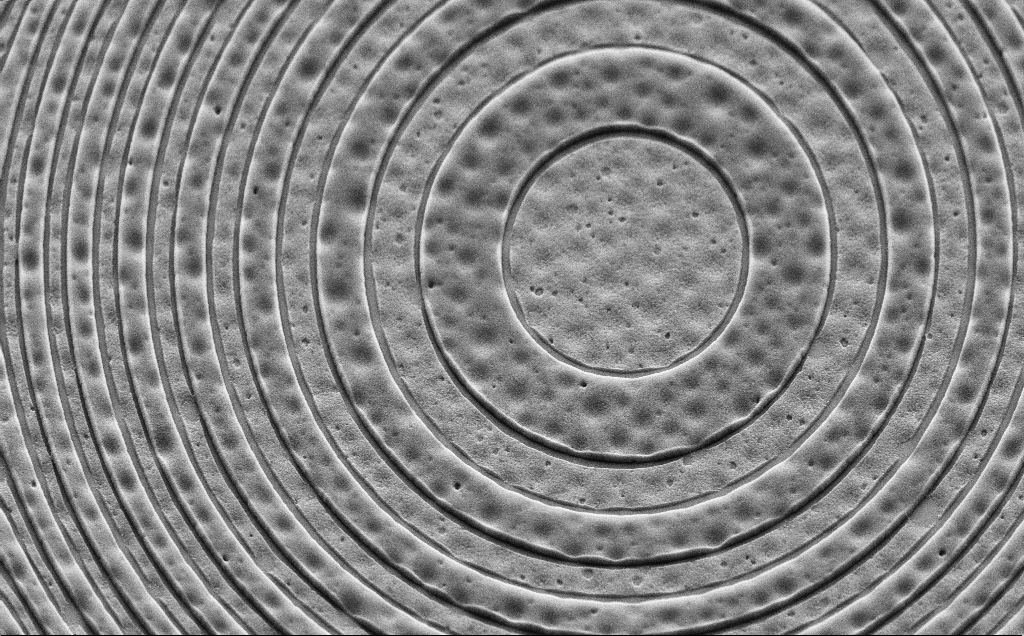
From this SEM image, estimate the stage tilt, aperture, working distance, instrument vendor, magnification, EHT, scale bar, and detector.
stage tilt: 45°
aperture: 30 µm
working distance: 6 mm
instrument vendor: Zeiss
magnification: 12.48 K X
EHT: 5 kV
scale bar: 1000 nm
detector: SE2